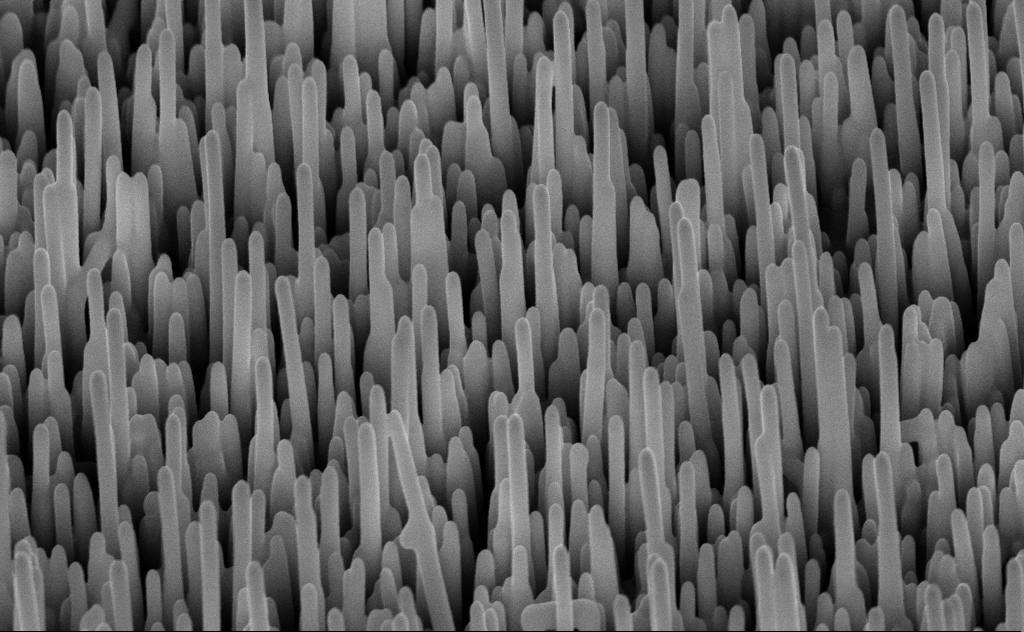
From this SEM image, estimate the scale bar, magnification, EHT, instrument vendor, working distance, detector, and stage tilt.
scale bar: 1000 nm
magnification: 40 K X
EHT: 10 kV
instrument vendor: Zeiss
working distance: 8 mm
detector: InLens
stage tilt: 45°